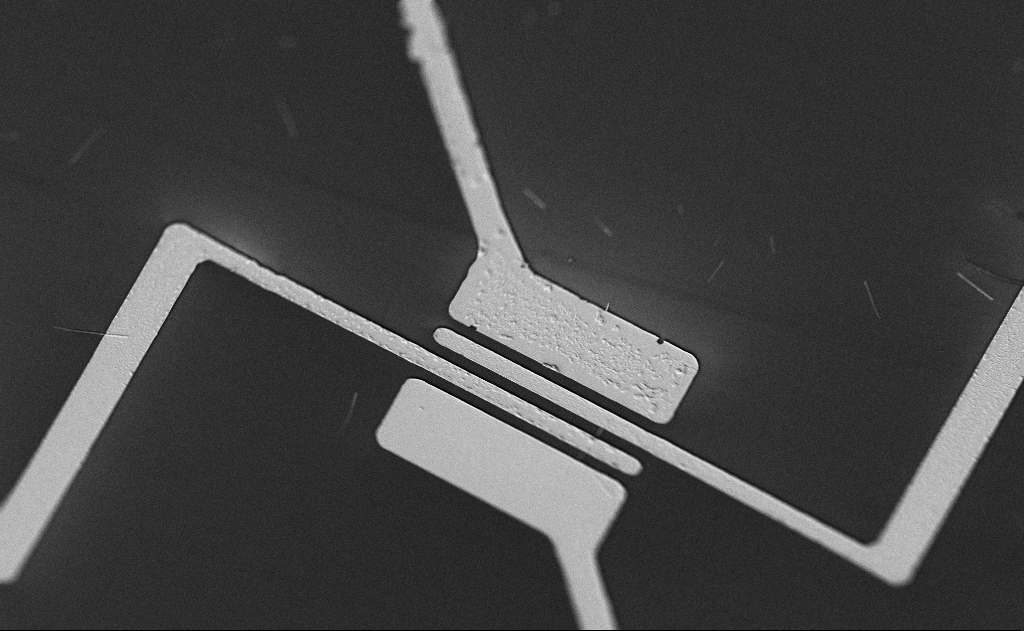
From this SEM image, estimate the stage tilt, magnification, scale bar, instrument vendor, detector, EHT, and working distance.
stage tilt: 0°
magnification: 3 K X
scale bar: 20000 nm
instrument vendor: Zeiss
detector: SE2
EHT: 5 kV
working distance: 21 mm